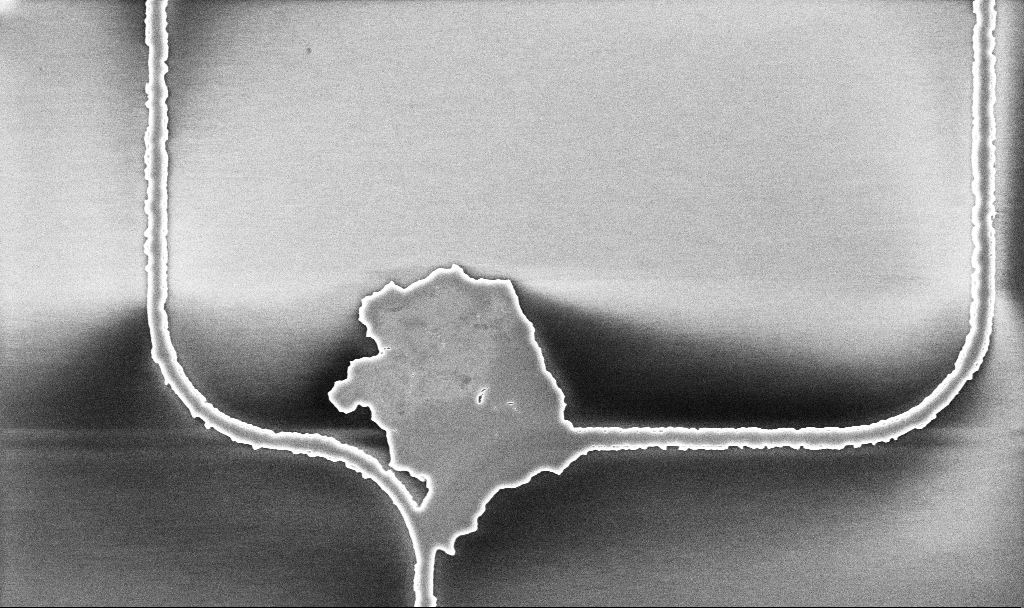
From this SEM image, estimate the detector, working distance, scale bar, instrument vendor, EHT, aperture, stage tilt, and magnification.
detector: InLens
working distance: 10.1 mm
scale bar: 2000 nm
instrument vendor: Zeiss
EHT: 5 kV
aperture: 30 µm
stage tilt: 0°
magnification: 10.55 K X